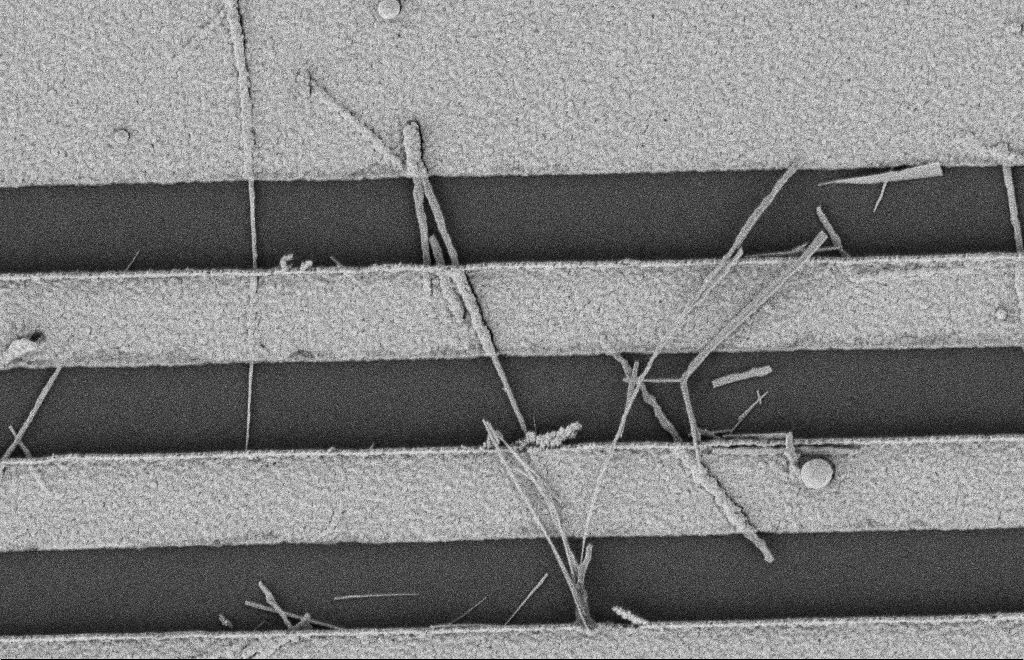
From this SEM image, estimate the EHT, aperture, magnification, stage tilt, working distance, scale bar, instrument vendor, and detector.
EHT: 2 kV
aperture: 20 µm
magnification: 16.33 K X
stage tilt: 0°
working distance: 12 mm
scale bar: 2000 nm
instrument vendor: Zeiss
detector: SE2